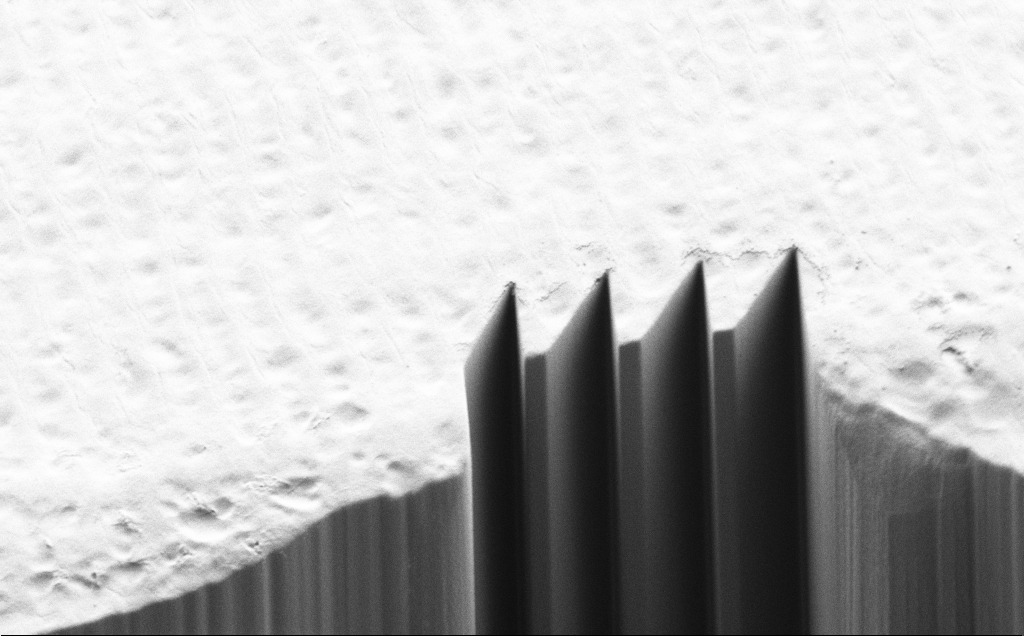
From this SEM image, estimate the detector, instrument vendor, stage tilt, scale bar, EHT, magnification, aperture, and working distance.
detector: SE2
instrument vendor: Zeiss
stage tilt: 45°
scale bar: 20000 nm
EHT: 0.9 kV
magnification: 2.98 K X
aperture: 30 µm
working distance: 7 mm